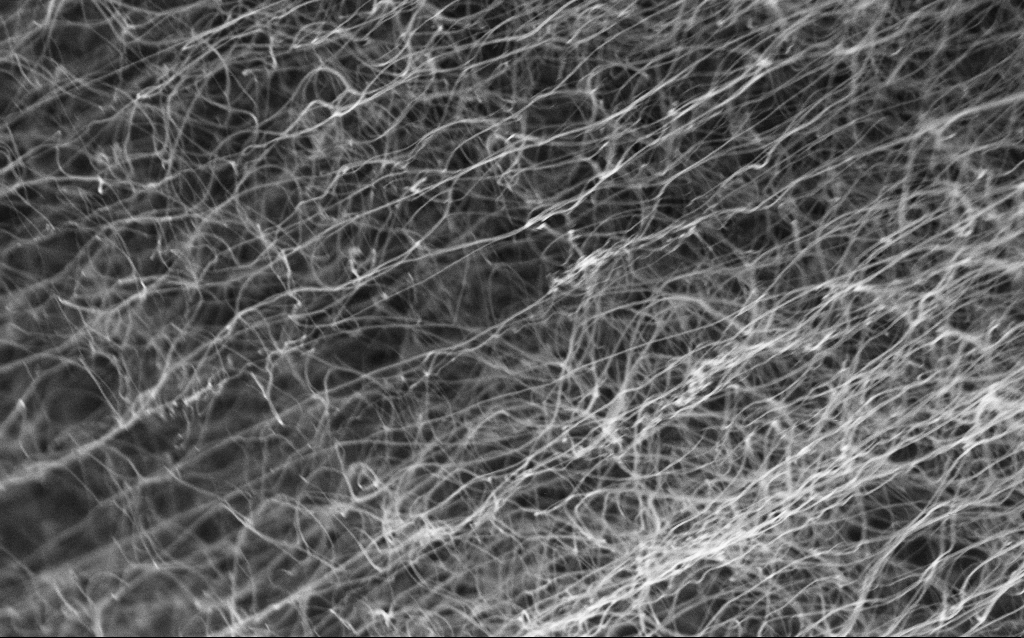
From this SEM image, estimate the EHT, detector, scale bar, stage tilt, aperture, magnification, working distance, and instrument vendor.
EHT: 5 kV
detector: InLens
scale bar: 1000 nm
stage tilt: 0°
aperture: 30 µm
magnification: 63.81 K X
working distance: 2.3 mm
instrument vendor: Zeiss